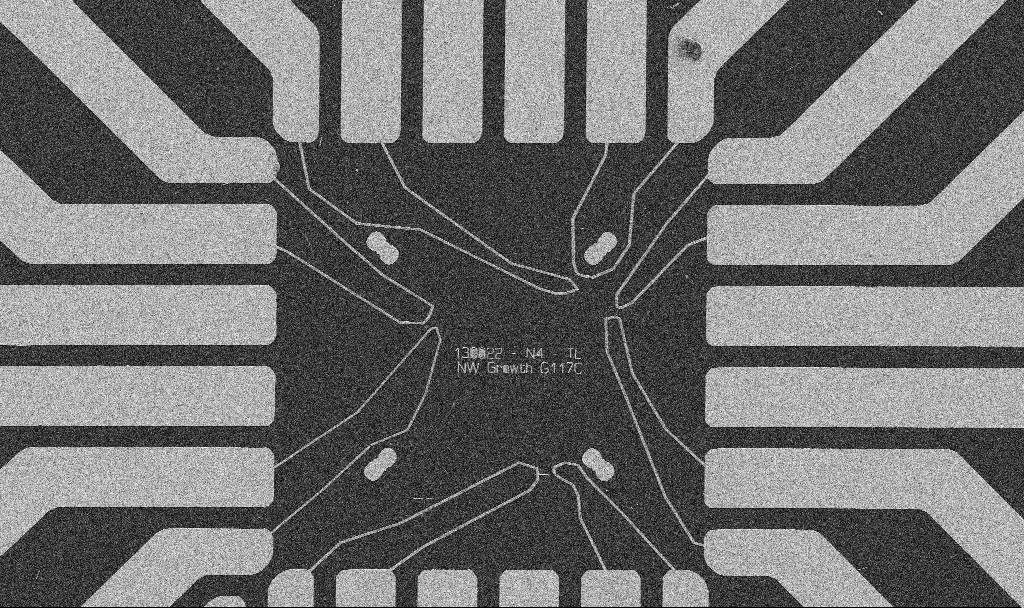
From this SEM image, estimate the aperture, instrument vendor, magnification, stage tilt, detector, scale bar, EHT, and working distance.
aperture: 30 µm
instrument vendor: Zeiss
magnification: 1 K X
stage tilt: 0°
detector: SE2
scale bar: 20000 nm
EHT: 5 kV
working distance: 8.7 mm